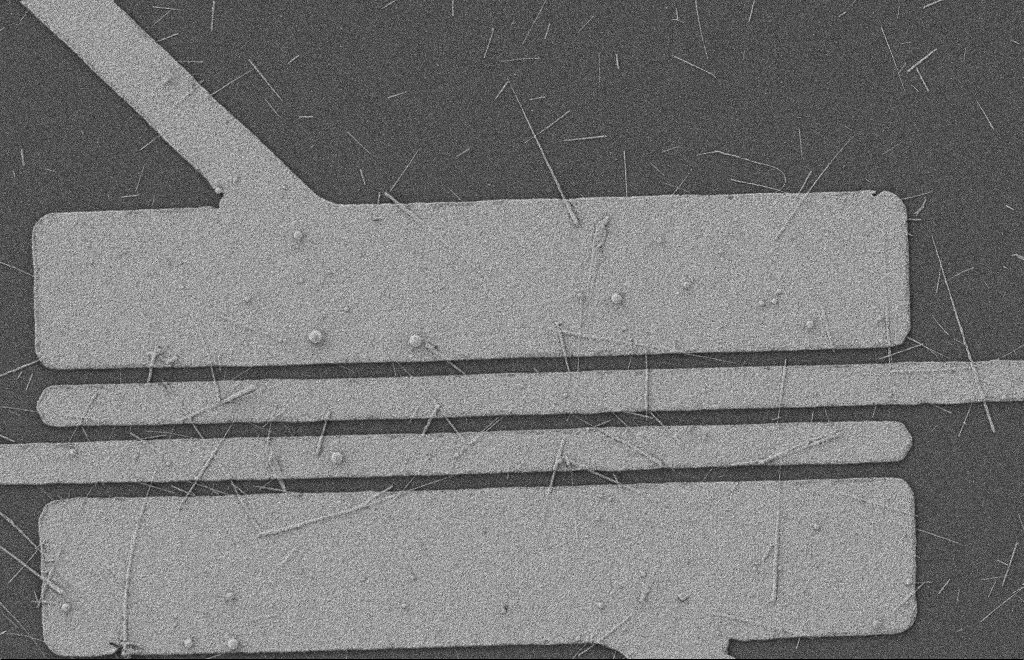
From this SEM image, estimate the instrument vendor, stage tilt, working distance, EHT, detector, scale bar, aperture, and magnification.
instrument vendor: Zeiss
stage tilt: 0°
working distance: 8 mm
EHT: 2 kV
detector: SE2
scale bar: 2000 nm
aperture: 20 µm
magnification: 5.24 K X